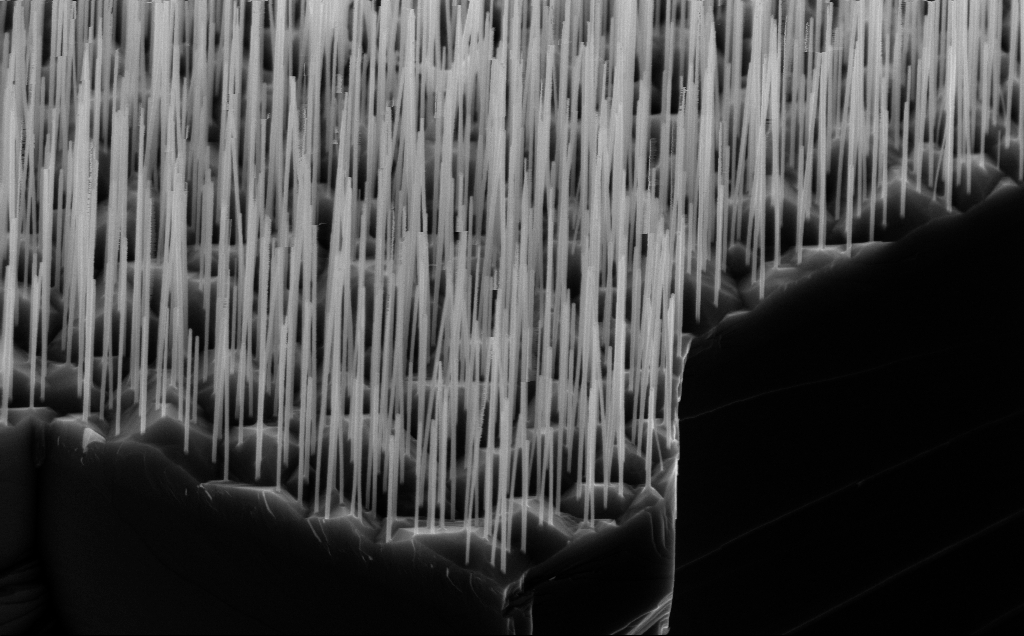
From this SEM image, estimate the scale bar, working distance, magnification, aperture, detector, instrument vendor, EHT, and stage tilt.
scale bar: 2000 nm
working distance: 5 mm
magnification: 35.06 K X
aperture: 30 µm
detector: InLens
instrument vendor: Zeiss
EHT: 10 kV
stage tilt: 45°